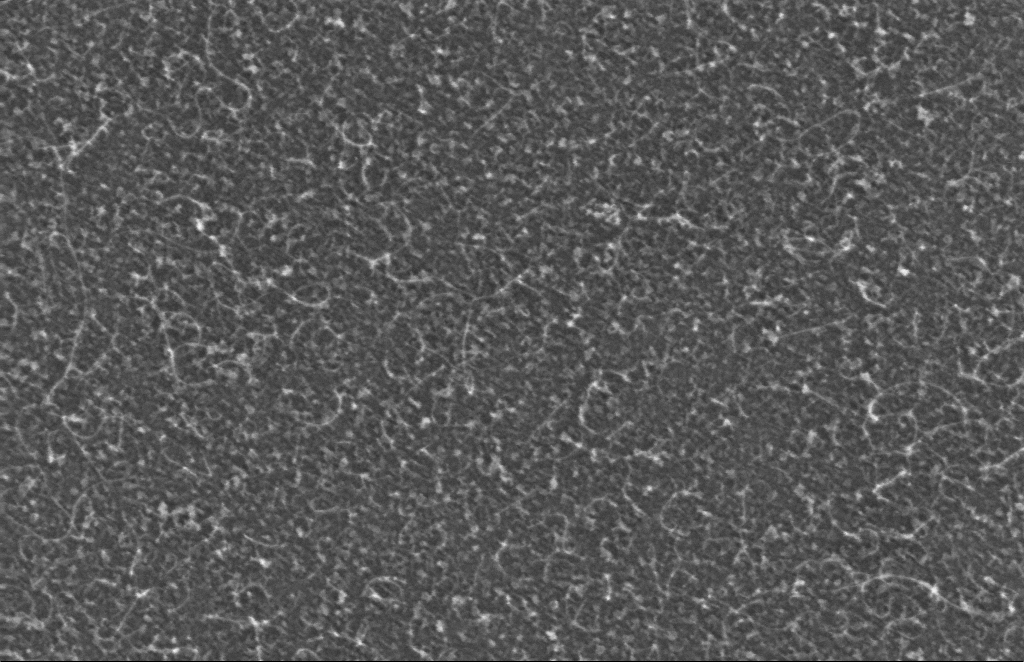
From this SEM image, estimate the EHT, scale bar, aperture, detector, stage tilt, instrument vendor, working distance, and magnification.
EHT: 5 kV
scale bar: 100 nm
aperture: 30 µm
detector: InLens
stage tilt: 0°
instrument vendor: Zeiss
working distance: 6 mm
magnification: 253.55 K X